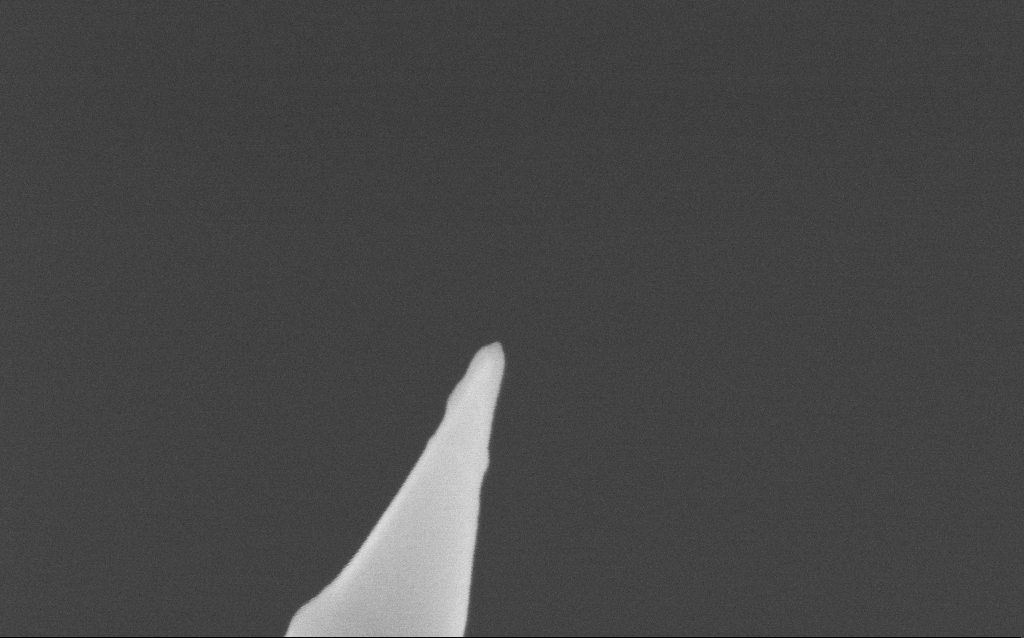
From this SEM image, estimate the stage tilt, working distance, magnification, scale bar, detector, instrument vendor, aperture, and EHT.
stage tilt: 0°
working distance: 5 mm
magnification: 250 K X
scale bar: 200 nm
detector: InLens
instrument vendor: Zeiss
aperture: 30 µm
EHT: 5 kV